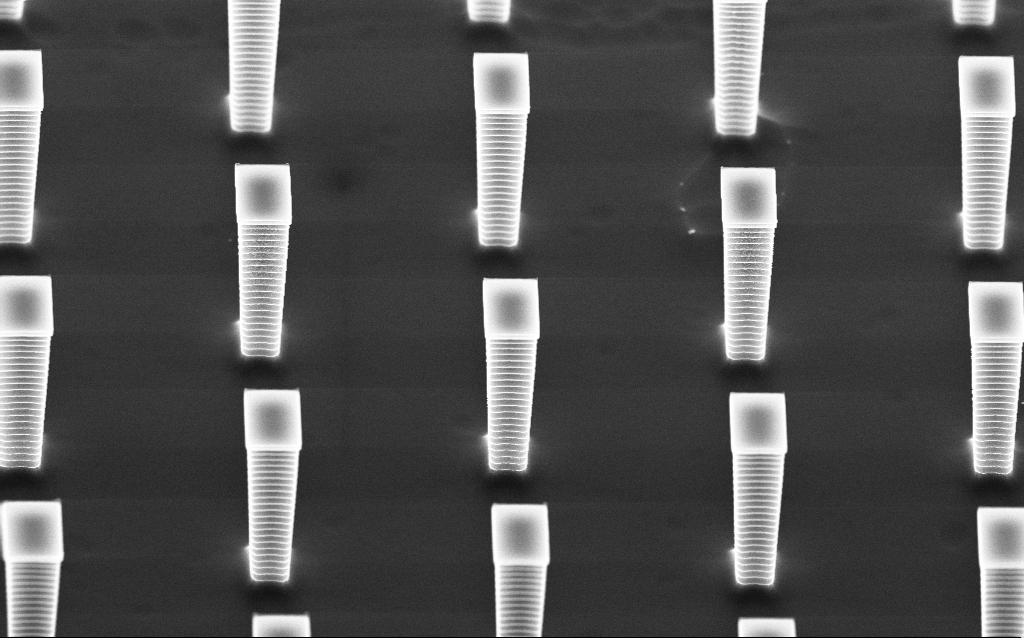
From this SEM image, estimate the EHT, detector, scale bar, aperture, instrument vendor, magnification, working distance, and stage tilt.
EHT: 10 kV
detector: InLens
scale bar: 2000 nm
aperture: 30 µm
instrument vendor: Zeiss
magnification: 9.89 K X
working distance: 4.8 mm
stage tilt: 45°